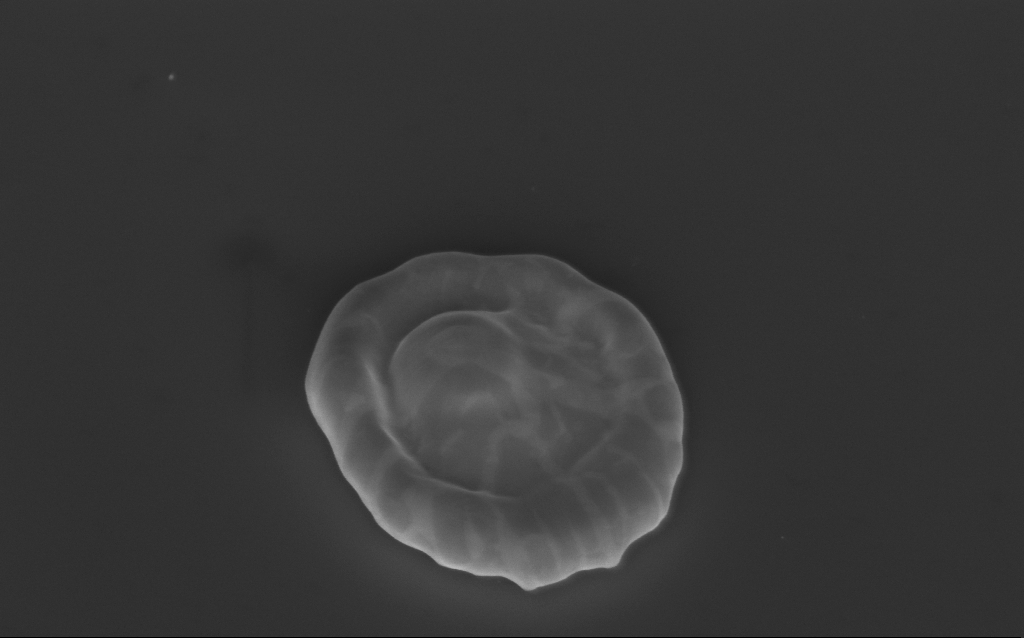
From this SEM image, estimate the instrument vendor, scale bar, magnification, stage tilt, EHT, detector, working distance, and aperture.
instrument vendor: Zeiss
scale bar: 1000 nm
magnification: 69.64 K X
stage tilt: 40°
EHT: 4 kV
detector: InLens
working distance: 5 mm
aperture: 30 µm